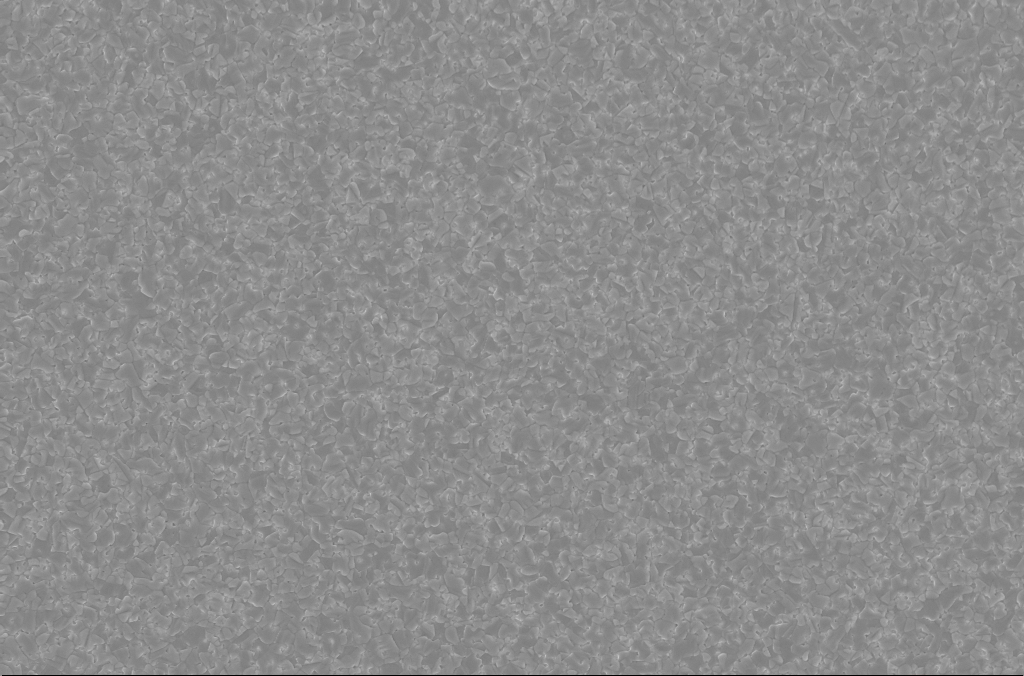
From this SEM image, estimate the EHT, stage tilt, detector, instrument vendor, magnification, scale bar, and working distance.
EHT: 5 kV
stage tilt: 0°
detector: InLens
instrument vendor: Zeiss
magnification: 10 K X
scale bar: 2000 nm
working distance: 3 mm